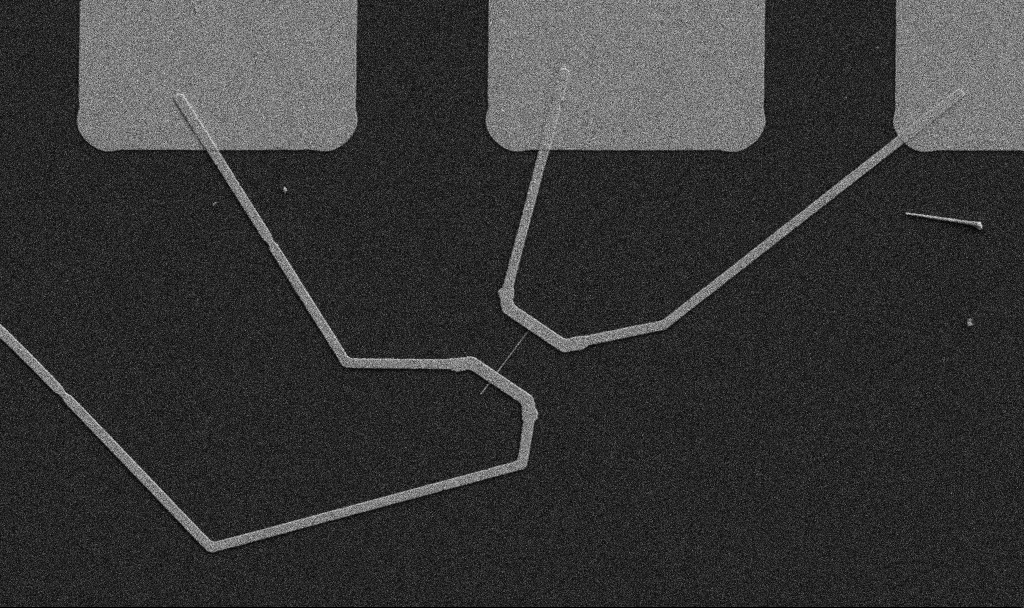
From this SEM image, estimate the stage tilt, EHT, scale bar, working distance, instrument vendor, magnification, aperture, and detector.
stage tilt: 0°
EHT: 5 kV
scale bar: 10000 nm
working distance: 10.7 mm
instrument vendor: Zeiss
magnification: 5 K X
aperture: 30 µm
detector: SE2